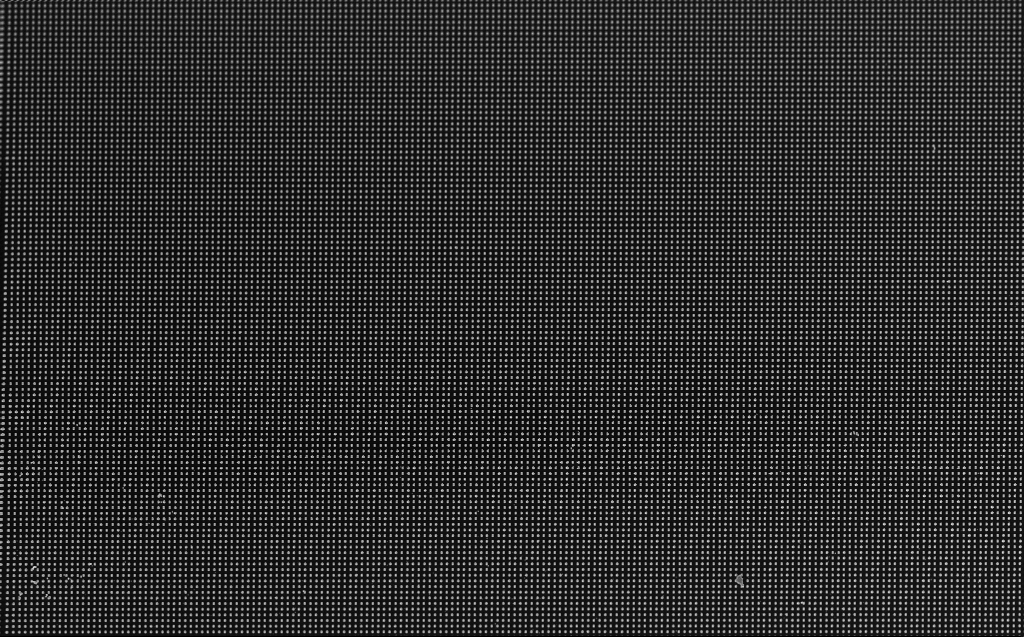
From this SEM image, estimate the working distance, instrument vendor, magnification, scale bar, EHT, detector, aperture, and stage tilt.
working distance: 6 mm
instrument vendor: Zeiss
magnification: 2 K X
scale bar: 10000 nm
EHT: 5 kV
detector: InLens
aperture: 30 µm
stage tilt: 30°